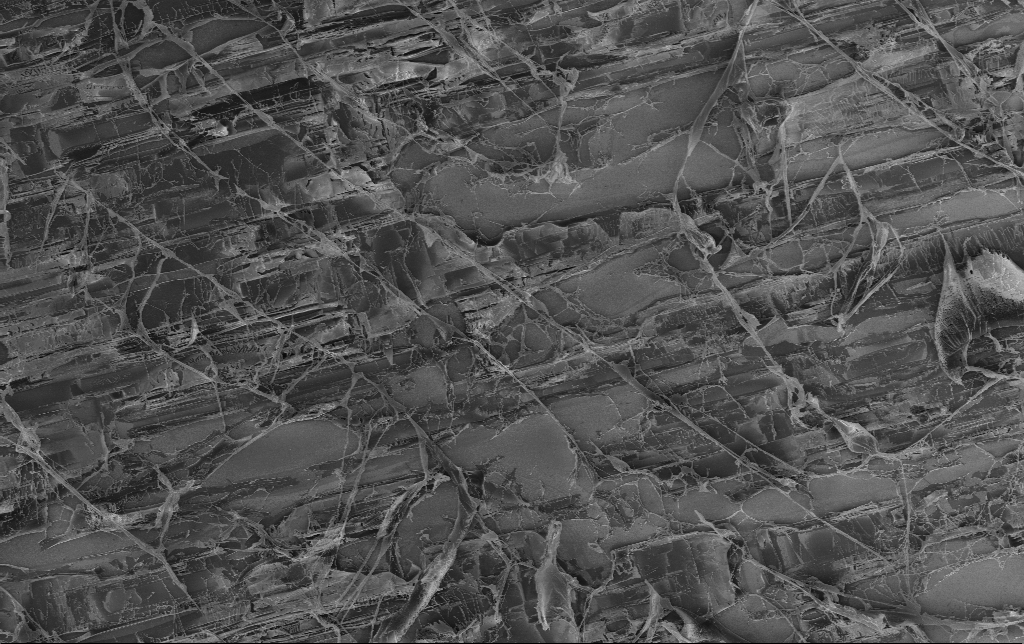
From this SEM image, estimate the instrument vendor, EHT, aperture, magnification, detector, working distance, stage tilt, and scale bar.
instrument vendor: Zeiss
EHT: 1.5 kV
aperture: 30 µm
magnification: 1.51 K X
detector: InLens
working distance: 3.4 mm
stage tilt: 0°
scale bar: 20000 nm